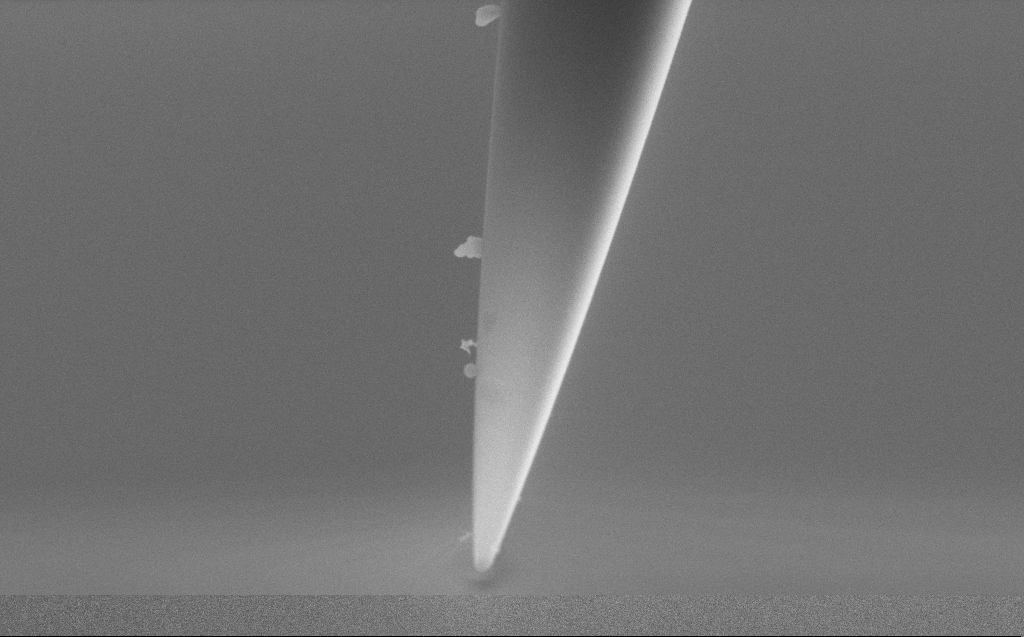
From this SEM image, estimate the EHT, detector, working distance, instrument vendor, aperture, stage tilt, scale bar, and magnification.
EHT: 5 kV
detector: SE2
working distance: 5 mm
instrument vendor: Zeiss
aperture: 30 µm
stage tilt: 45.1°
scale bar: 1000 nm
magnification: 46.53 K X